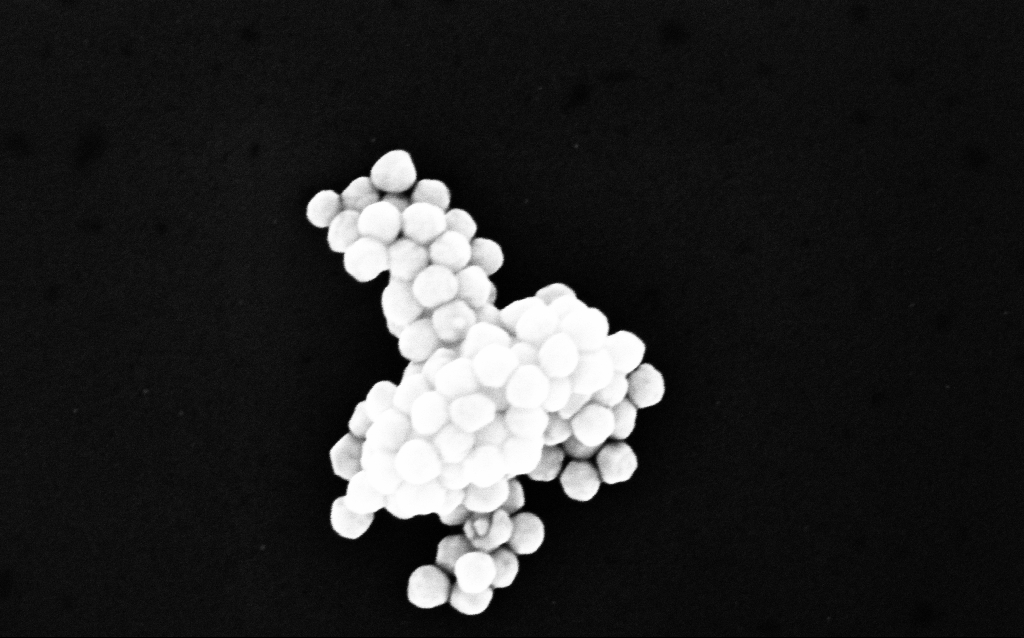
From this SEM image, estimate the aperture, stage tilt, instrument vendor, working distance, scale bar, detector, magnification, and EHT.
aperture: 30 µm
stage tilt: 0°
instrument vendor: Zeiss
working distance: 3.1 mm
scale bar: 100 nm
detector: InLens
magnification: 256.42 K X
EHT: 10 kV